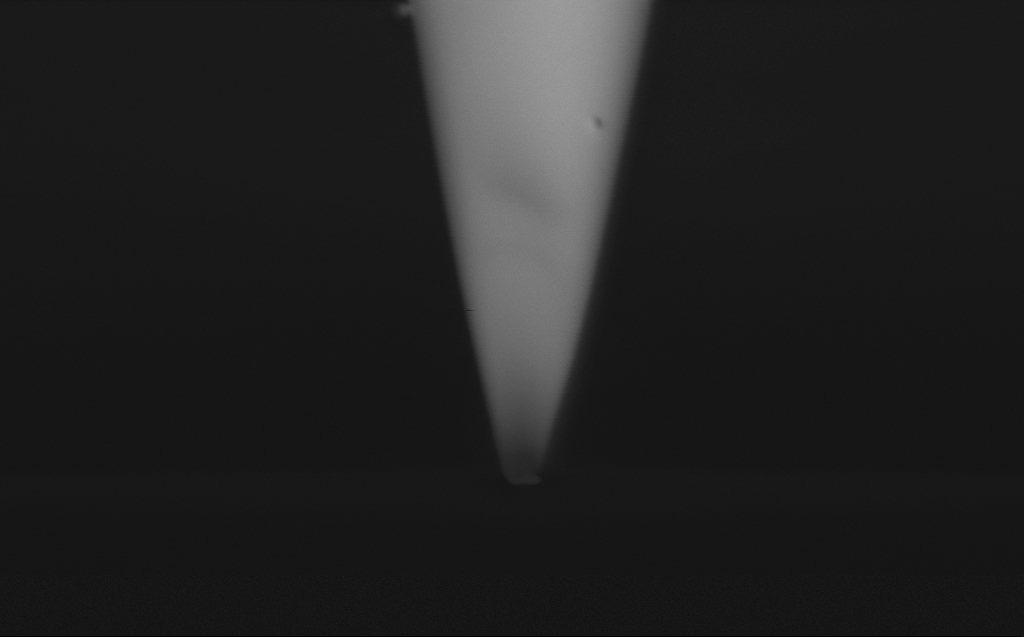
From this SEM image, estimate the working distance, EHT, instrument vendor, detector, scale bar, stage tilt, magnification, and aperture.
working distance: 3 mm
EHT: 1 kV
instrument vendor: Zeiss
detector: InLens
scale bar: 200 nm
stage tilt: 45.1°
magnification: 135.4 K X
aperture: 30 µm